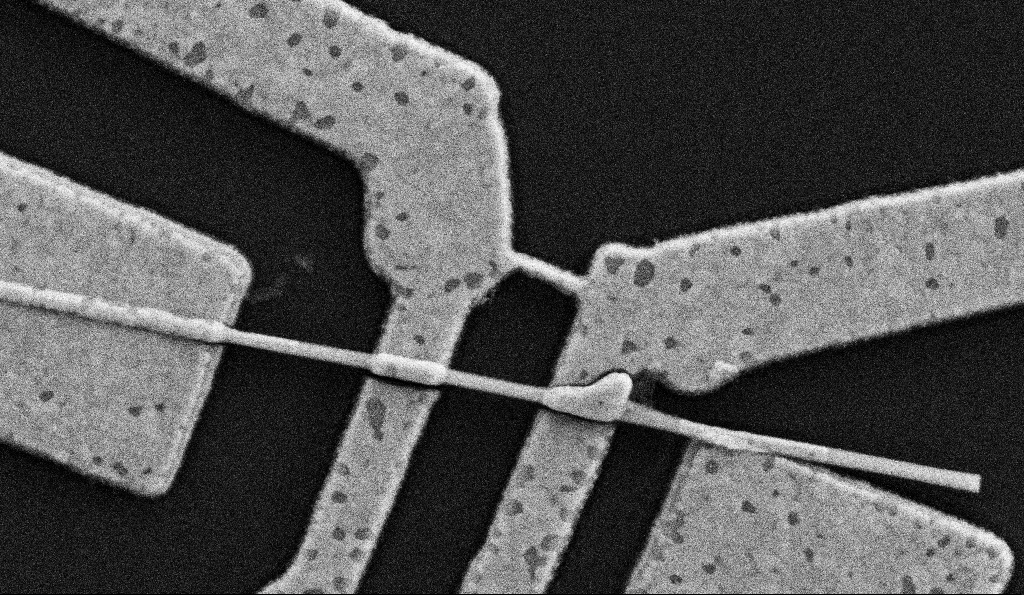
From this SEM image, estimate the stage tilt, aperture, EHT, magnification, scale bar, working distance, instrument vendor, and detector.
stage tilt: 0°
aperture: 30 µm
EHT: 5 kV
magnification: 62.47 K X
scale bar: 1000 nm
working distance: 8.5 mm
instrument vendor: Zeiss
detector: SE2